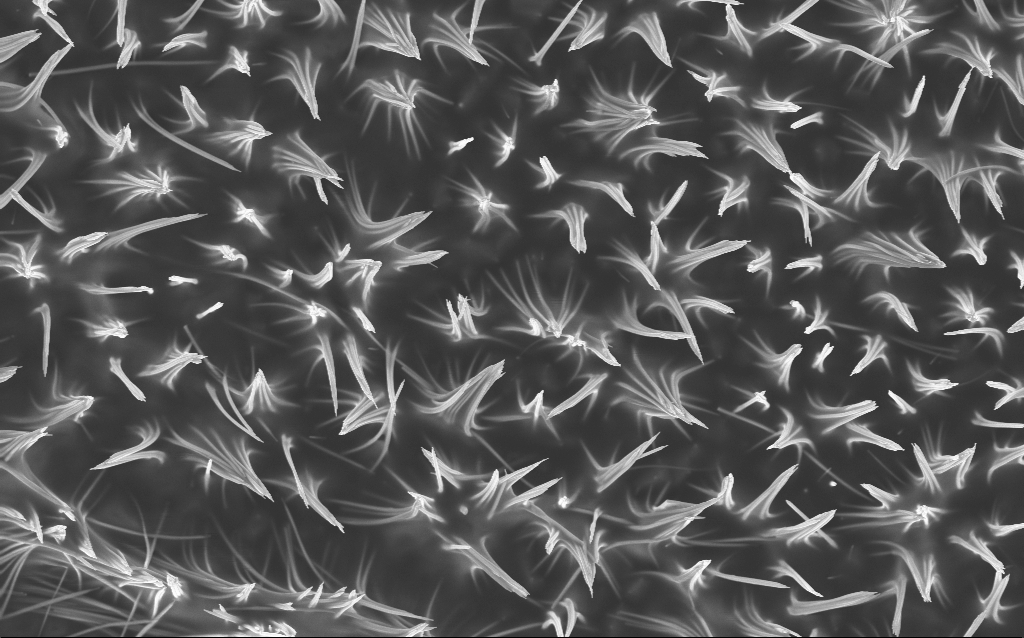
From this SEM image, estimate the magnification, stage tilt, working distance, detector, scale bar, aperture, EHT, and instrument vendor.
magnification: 14.36 K X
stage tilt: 0°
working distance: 3.1 mm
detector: InLens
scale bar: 1000 nm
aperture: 30 µm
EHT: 5 kV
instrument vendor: Zeiss